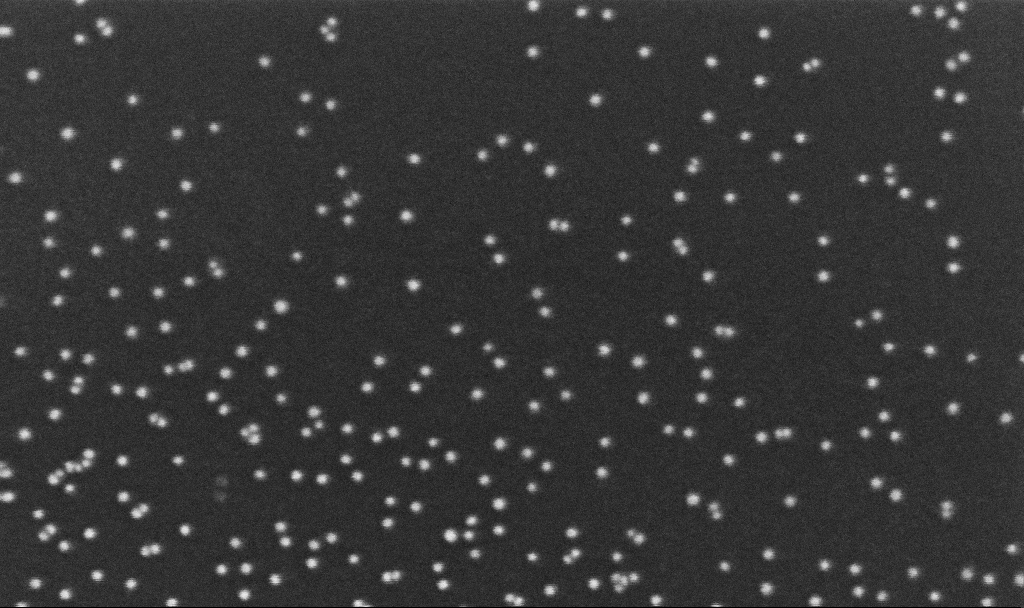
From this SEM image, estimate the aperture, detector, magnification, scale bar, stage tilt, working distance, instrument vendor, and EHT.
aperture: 30 µm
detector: InLens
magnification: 350 K X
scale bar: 200 nm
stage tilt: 0°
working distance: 3.2 mm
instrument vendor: Zeiss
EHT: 10 kV